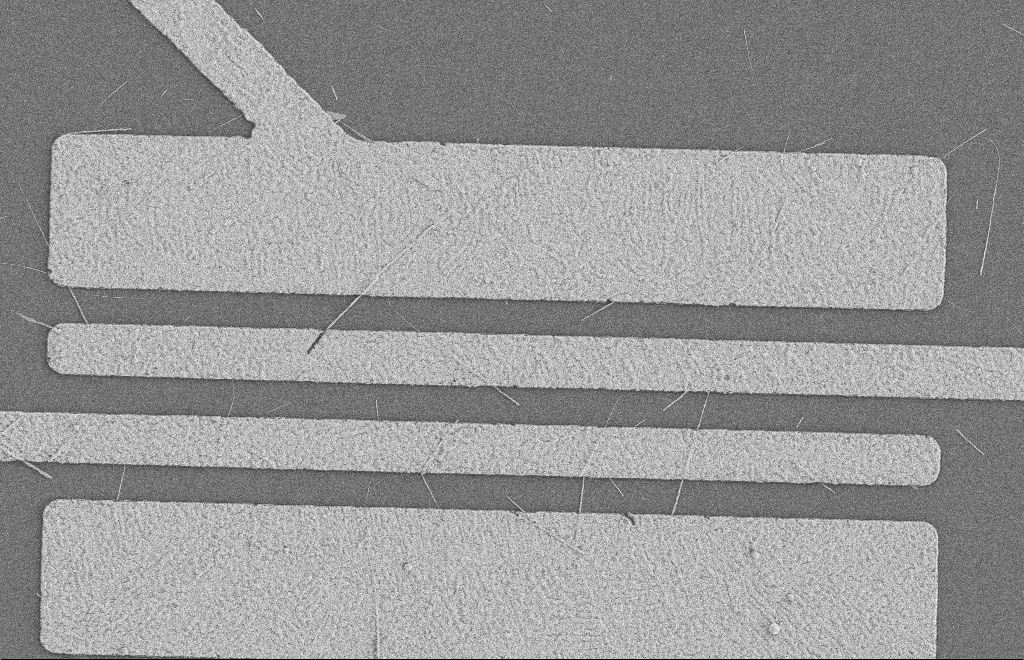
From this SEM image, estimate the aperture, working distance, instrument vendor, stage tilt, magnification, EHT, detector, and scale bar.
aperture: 20 µm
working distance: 8 mm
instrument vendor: Zeiss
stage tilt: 0°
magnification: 5.39 K X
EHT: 2 kV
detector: SE2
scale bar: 2000 nm